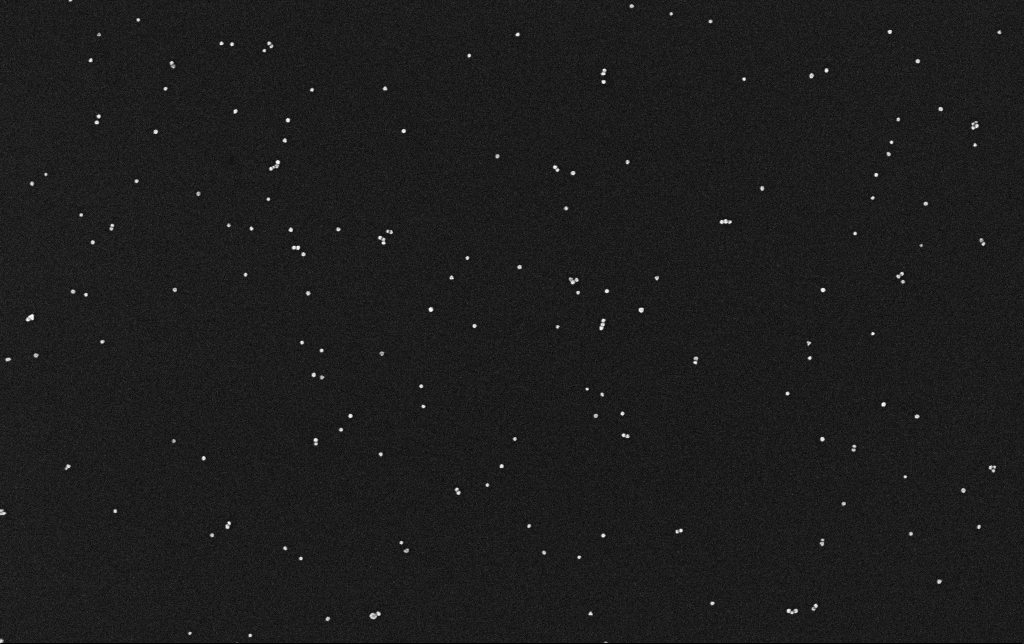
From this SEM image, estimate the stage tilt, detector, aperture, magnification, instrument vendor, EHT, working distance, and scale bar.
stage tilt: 0°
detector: InLens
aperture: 30 µm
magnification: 100 K X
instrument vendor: Zeiss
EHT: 10 kV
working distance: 3.1 mm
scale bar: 200 nm